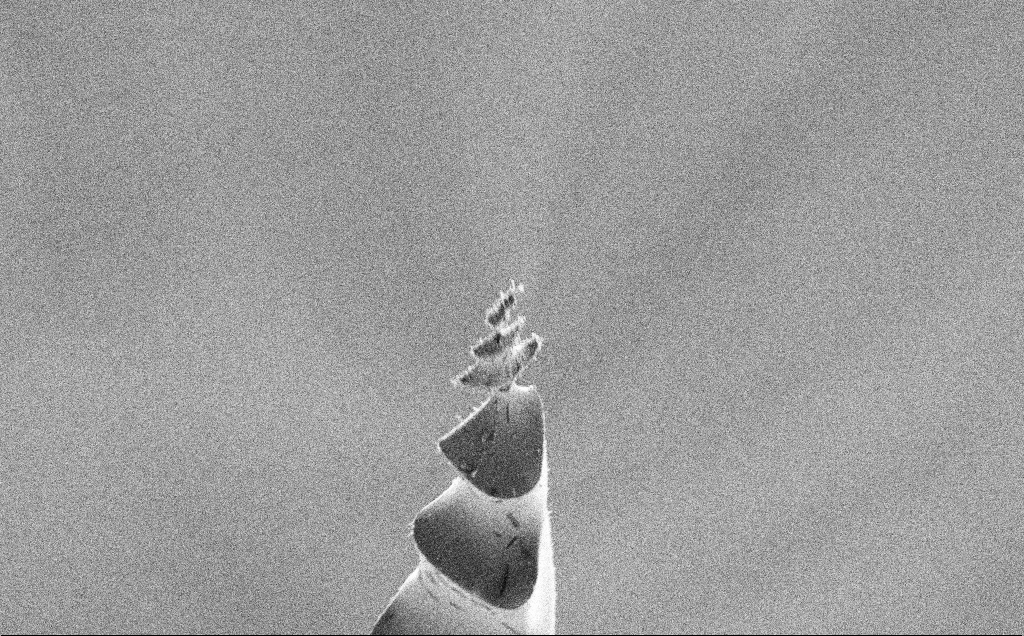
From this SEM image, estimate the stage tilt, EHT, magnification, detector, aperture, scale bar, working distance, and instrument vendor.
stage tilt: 20°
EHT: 5 kV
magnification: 2.19 K X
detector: SE2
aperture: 30 µm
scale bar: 10000 nm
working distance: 4 mm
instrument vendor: Zeiss